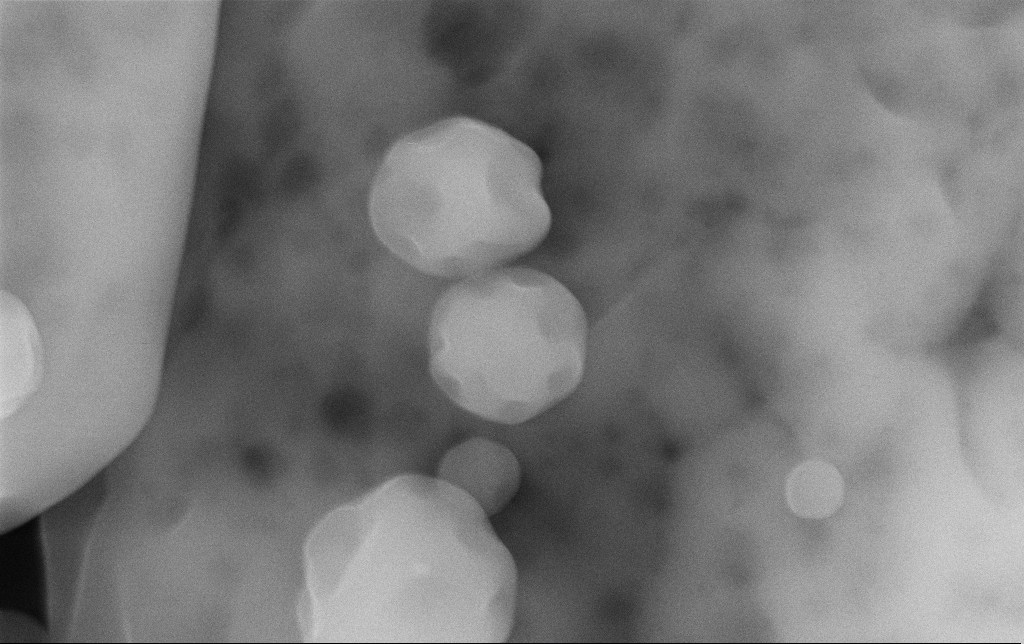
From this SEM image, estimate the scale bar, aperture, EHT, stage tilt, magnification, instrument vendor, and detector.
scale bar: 200 nm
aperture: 30 µm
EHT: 15 kV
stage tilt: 0°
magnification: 124 K X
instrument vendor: Zeiss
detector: InLens